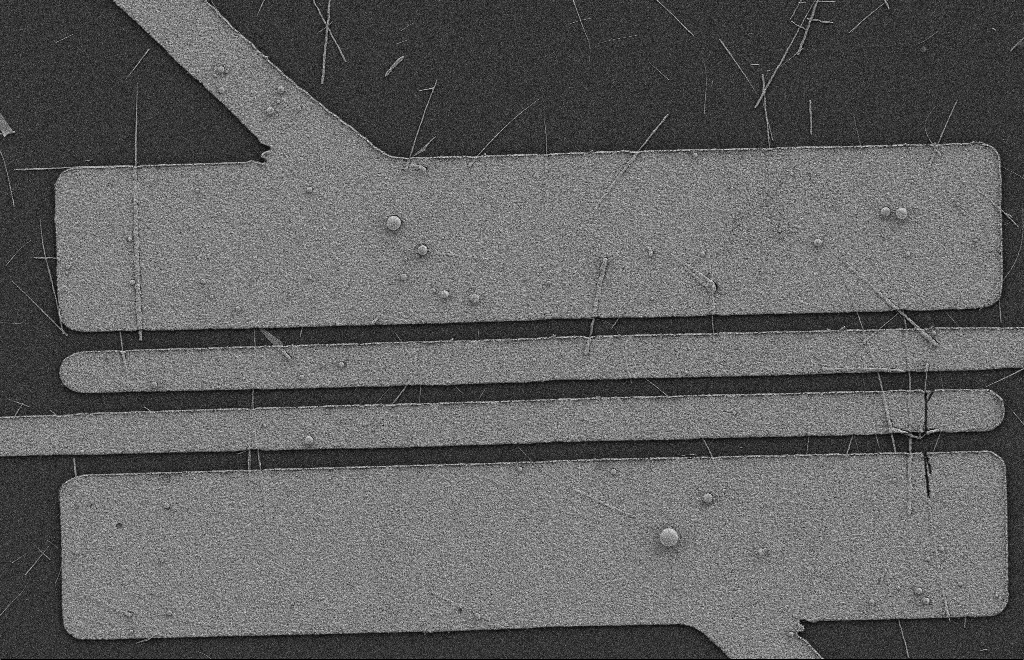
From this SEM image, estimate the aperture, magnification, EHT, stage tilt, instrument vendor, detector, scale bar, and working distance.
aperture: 20 µm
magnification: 5.67 K X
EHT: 2 kV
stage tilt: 0°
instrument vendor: Zeiss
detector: SE2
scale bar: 2000 nm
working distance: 9 mm